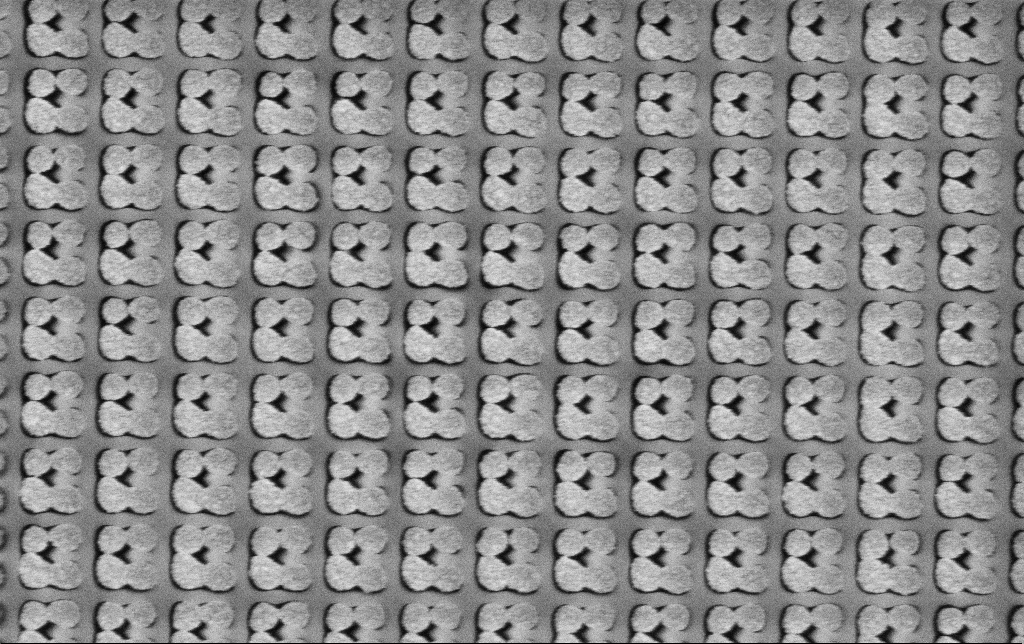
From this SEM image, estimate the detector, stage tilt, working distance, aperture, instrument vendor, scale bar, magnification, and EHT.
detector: SE2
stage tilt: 0°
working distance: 6.1 mm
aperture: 30 µm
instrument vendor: Zeiss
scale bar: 1000 nm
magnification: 60.7 K X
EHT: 3 kV